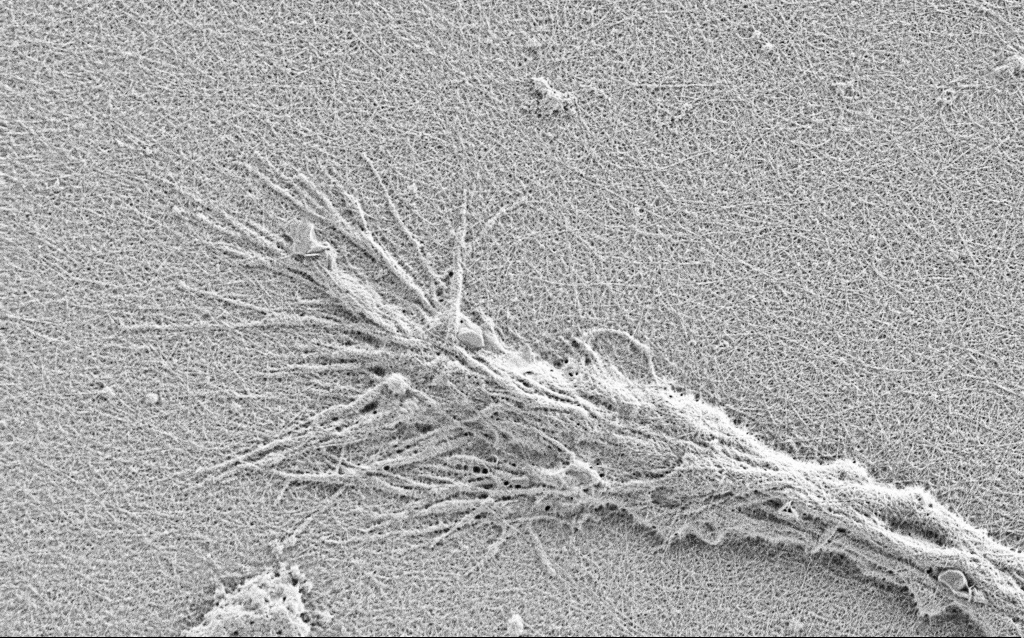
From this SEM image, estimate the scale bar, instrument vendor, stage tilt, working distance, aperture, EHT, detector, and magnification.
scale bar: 2000 nm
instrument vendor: Zeiss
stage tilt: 0°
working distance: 3 mm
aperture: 30 µm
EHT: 0.9 kV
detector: SE2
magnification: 10 K X